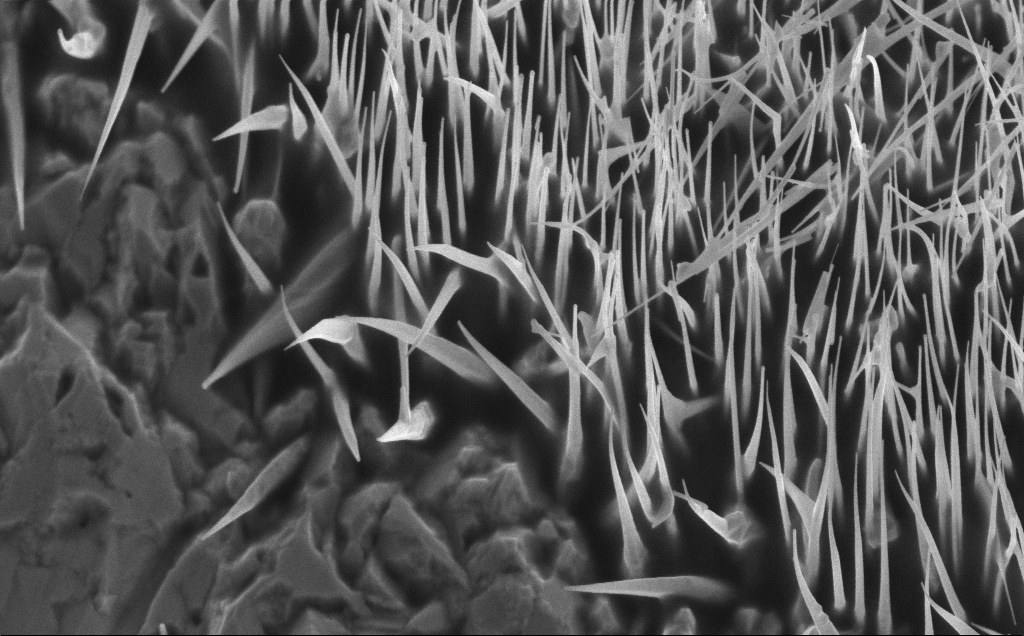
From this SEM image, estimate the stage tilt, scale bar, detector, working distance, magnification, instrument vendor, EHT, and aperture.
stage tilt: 30°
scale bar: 1000 nm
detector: InLens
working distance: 7 mm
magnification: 24.4 K X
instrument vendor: Zeiss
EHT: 5 kV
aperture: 30 µm